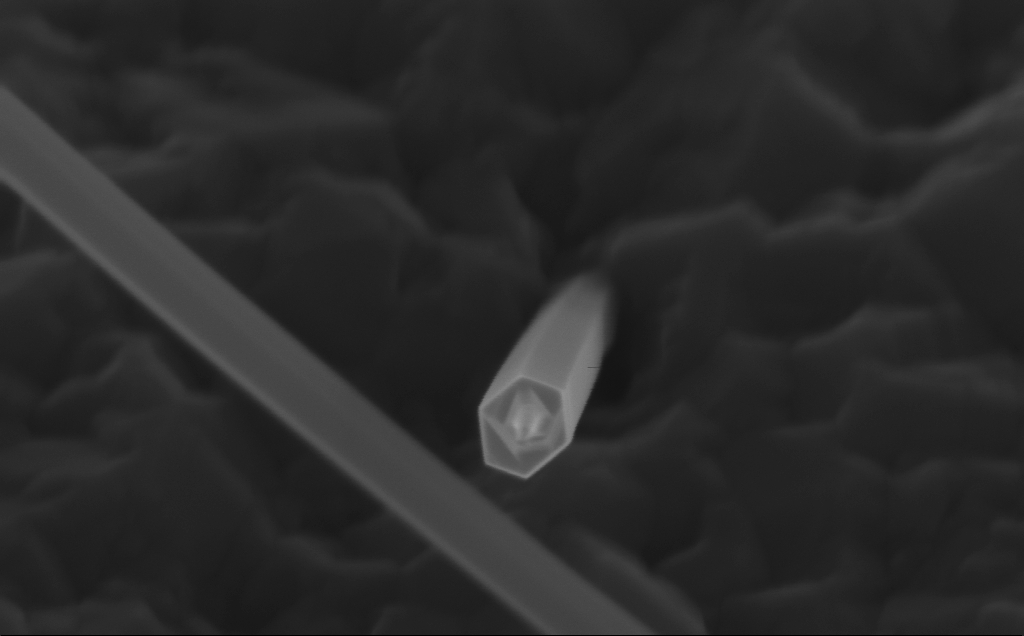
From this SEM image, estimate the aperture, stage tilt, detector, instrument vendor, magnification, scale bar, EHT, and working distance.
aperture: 30 µm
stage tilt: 45°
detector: InLens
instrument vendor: Zeiss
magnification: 231.03 K X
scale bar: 200 nm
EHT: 10 kV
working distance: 5 mm